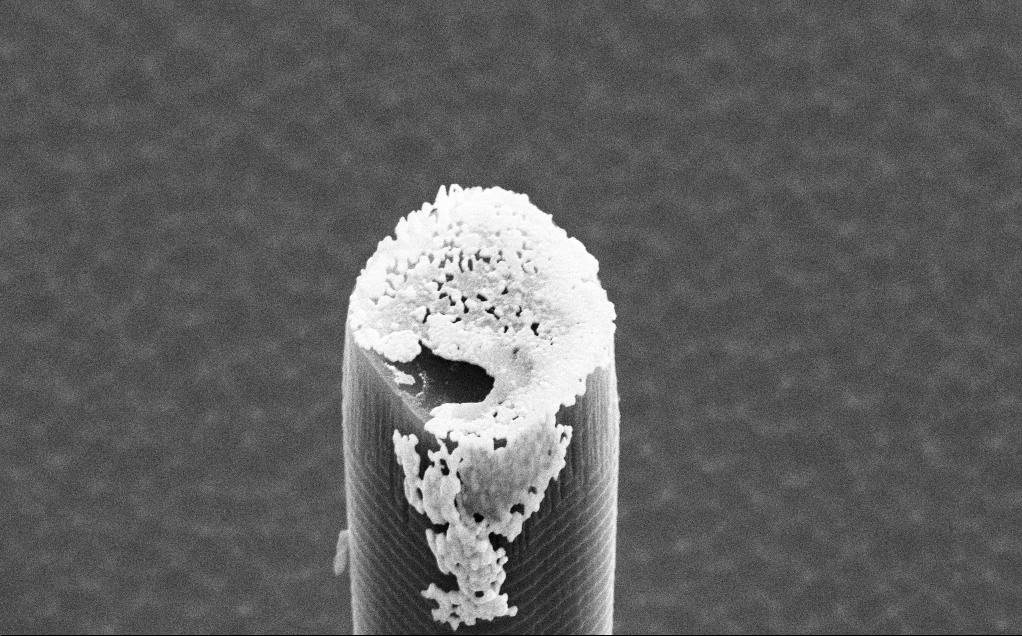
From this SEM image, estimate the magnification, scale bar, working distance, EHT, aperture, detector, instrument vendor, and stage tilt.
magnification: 14.07 K X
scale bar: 2000 nm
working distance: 10 mm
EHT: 15 kV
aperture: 30 µm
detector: SE2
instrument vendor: Zeiss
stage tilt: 50°